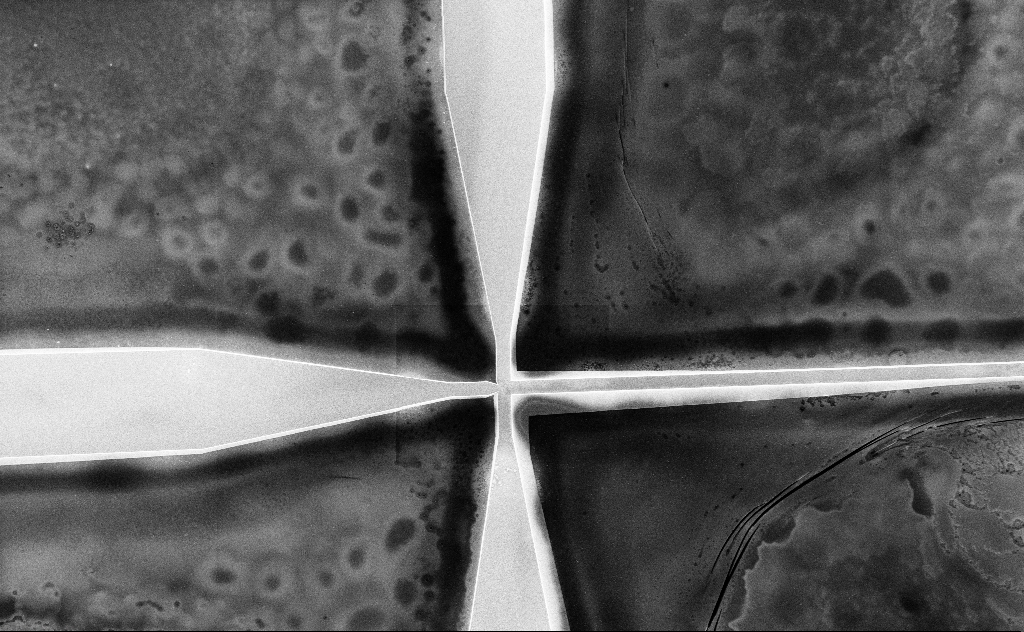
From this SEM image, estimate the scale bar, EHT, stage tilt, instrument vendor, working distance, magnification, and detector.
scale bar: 100000 nm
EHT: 5 kV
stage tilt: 0°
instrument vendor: Zeiss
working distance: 6 mm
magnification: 0.458 K X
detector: InLens